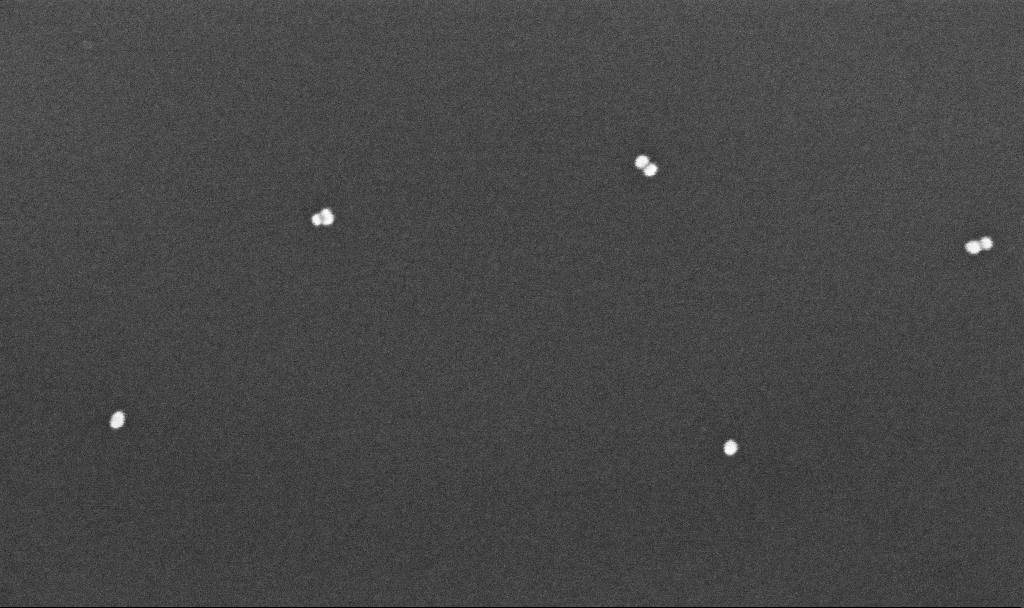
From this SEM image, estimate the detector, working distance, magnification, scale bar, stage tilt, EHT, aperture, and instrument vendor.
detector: InLens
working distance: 4.3 mm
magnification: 281.86 K X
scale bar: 200 nm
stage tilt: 0°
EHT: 10 kV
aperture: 30 µm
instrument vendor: Zeiss